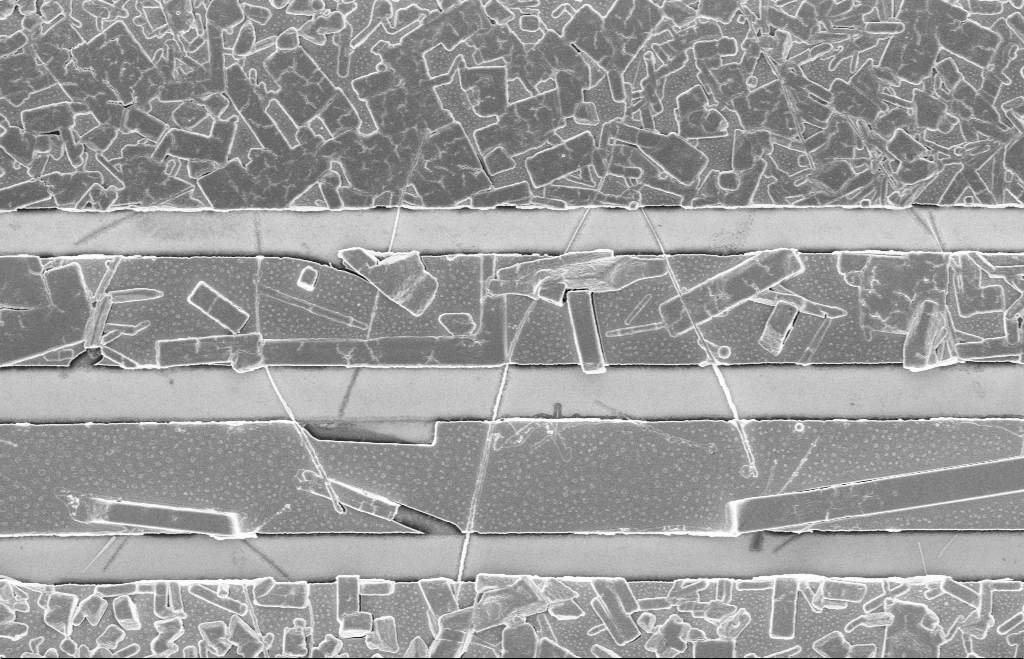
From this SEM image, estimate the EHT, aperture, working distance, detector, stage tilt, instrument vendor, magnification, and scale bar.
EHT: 5 kV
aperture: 20 µm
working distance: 8 mm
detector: InLens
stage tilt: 0°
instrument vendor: Zeiss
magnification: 10.08 K X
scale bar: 2000 nm